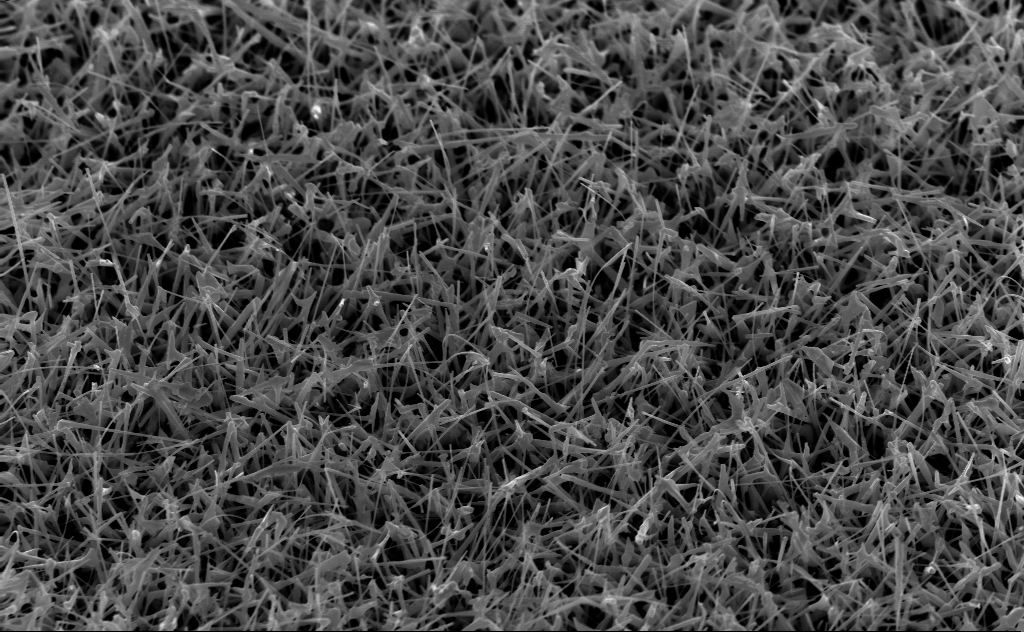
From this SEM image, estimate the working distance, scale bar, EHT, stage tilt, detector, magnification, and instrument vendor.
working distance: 5 mm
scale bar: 2000 nm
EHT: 10 kV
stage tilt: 45°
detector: InLens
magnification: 20 K X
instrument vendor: Zeiss